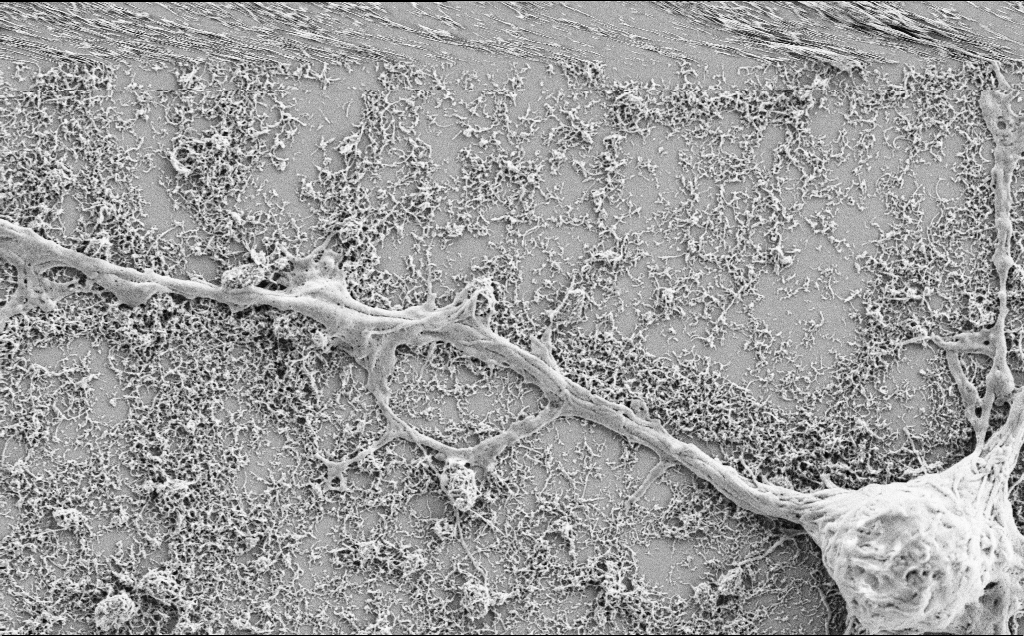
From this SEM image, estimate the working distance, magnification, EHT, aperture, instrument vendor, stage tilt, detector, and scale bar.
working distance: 7.1 mm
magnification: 10 K X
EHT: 2 kV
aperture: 30 µm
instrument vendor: Zeiss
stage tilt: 0°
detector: SE2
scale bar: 2000 nm